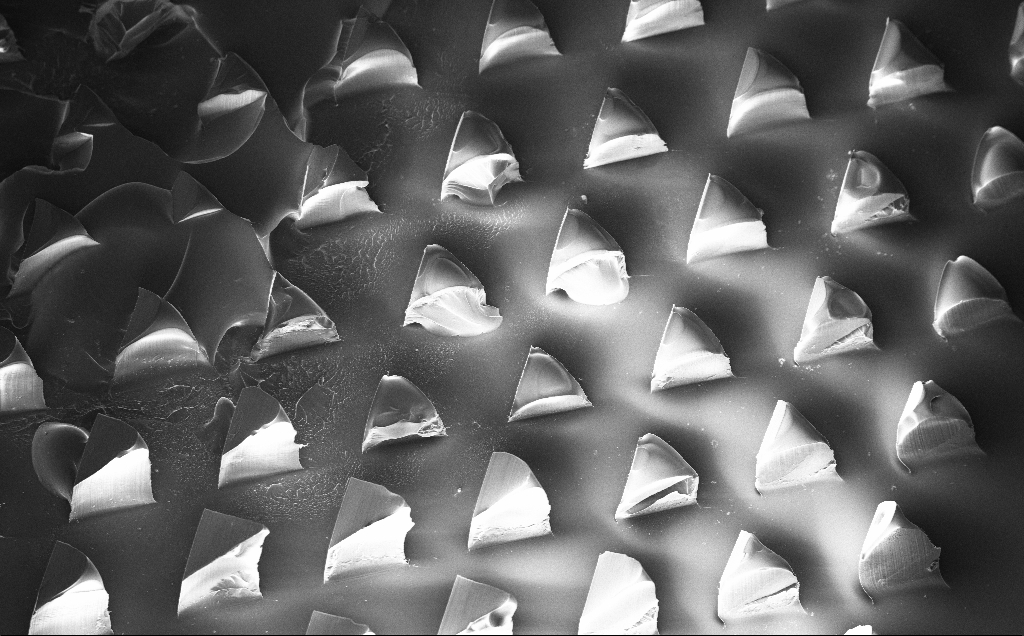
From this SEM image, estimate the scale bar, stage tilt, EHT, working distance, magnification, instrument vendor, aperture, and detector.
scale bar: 200000 nm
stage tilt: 20°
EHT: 10 kV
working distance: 9 mm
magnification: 0.121 K X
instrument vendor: Zeiss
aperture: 30 µm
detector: InLens